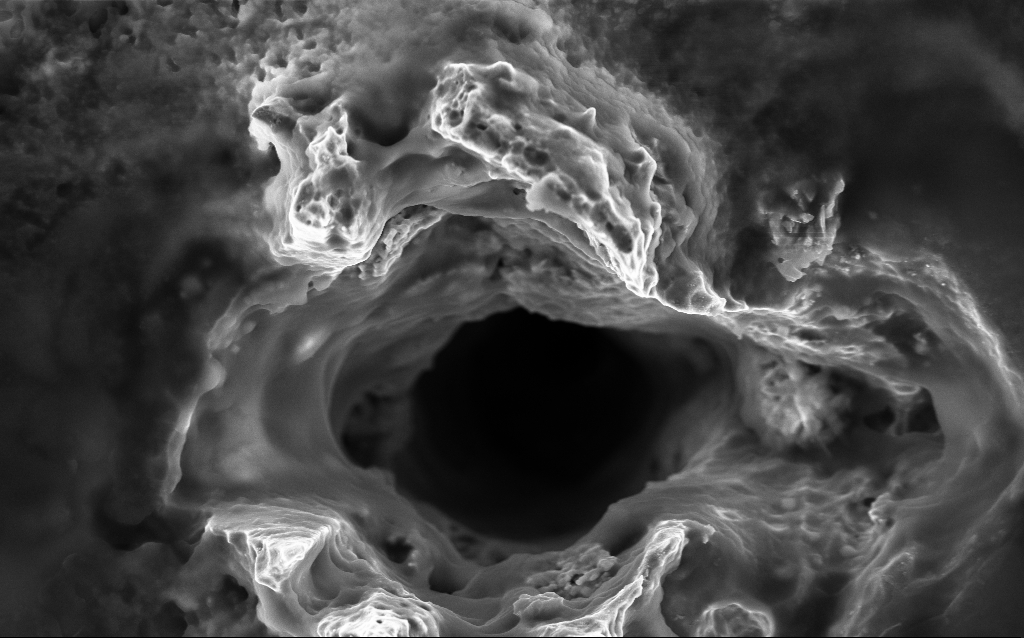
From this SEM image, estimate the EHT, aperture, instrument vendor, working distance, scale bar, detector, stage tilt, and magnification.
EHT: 5 kV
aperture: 30 µm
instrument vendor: Zeiss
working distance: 4.6 mm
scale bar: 2000 nm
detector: InLens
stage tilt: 0°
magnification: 20.35 K X